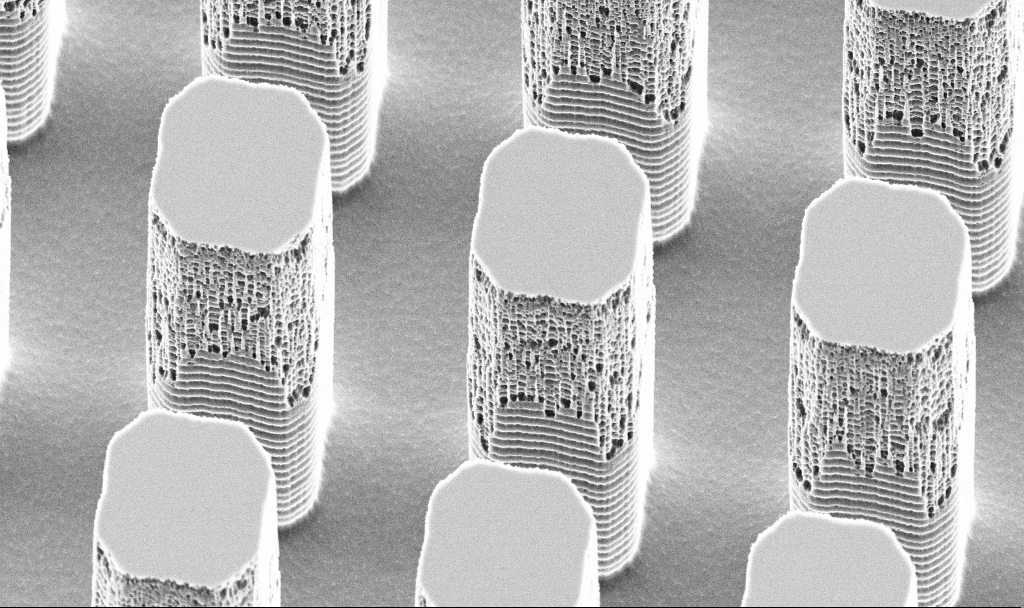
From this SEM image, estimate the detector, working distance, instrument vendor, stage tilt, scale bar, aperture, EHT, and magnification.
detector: SE2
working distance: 16.6 mm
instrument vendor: Zeiss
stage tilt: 45°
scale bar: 10000 nm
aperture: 30 µm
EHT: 5 kV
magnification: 5.94 K X